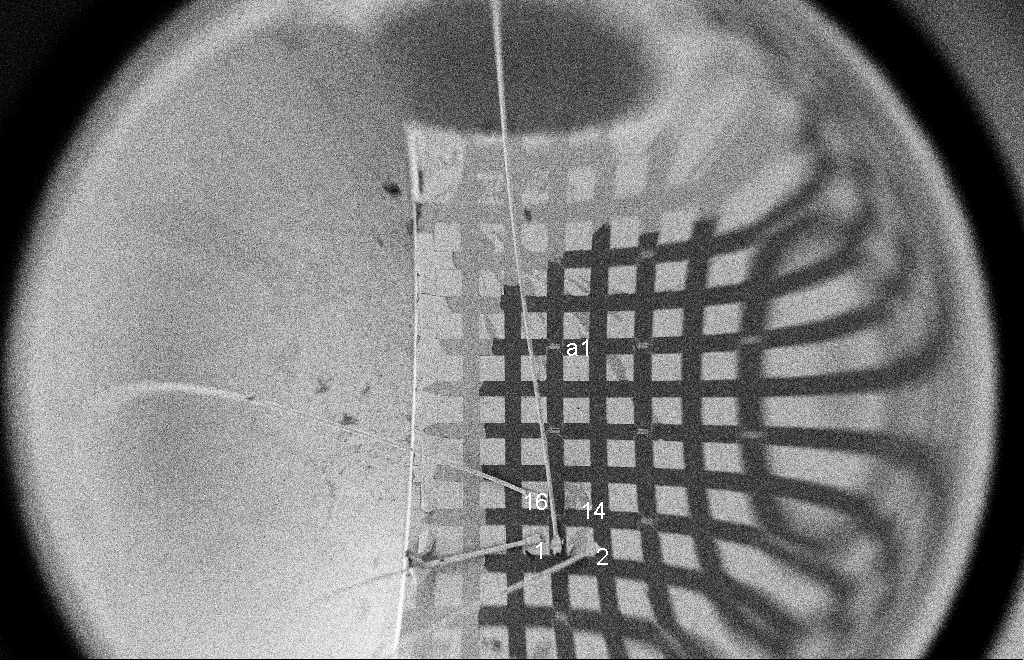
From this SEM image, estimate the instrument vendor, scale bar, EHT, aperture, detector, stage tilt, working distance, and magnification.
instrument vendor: Zeiss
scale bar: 200000 nm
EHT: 2 kV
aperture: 20 µm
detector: SE2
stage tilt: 0°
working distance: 9 mm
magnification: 0.064 K X